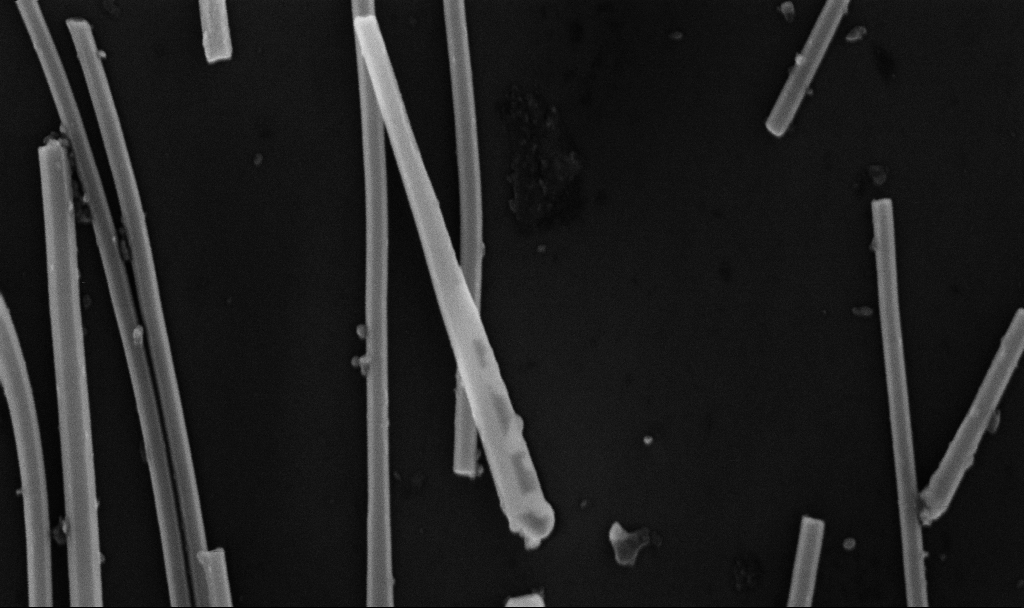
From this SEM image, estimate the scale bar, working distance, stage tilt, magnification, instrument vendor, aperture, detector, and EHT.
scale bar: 200 nm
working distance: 6.7 mm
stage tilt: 0°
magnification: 83.51 K X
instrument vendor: Zeiss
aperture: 30 µm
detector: InLens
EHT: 10 kV